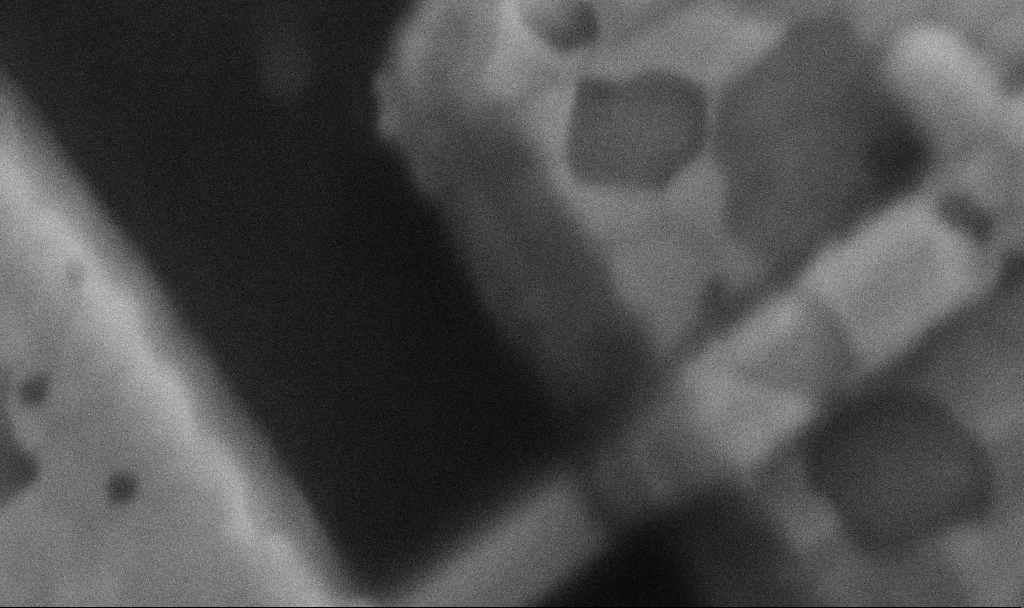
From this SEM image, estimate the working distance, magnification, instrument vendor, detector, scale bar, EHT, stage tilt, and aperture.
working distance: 8.5 mm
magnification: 200 K X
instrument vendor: Zeiss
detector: SE2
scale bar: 100 nm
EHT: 5 kV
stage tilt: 0°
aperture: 30 µm